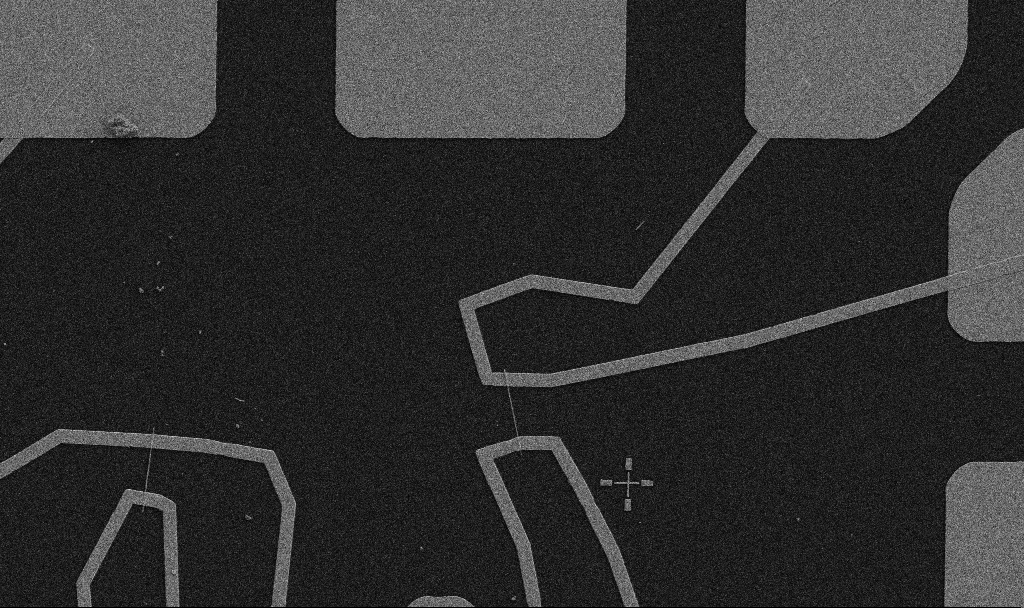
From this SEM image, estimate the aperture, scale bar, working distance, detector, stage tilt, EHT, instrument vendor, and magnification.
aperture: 30 µm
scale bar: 10000 nm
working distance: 10.7 mm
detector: SE2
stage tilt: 0°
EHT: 5 kV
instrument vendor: Zeiss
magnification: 5 K X